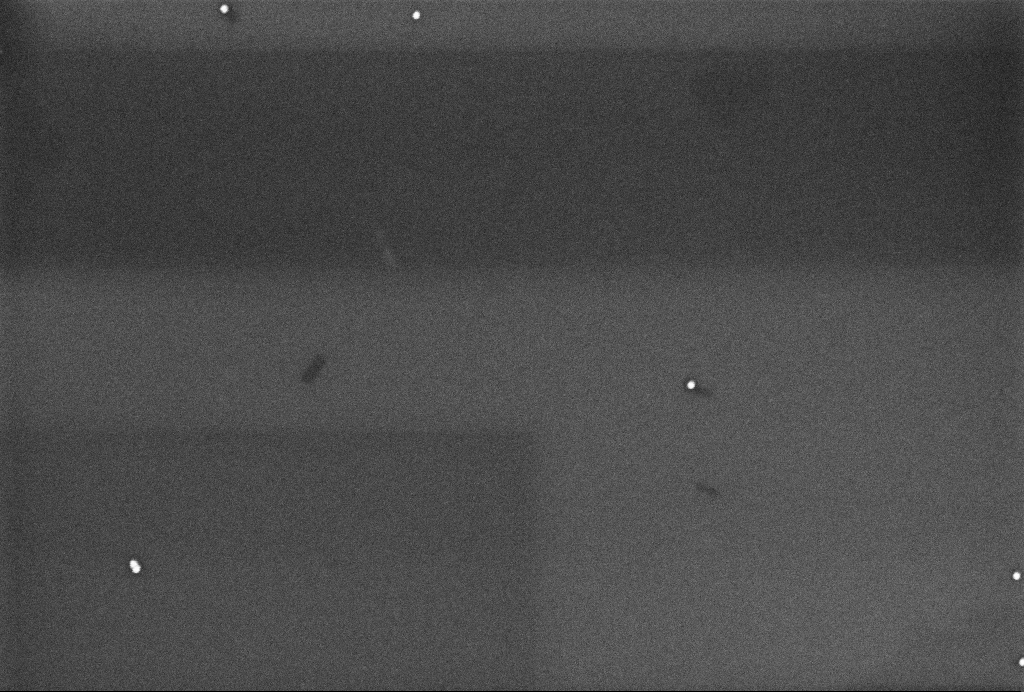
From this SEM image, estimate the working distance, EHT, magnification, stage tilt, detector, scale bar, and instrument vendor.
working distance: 3.2 mm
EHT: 3 kV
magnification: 99.83 K X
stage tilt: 0°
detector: InLens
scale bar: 200 nm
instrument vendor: Zeiss